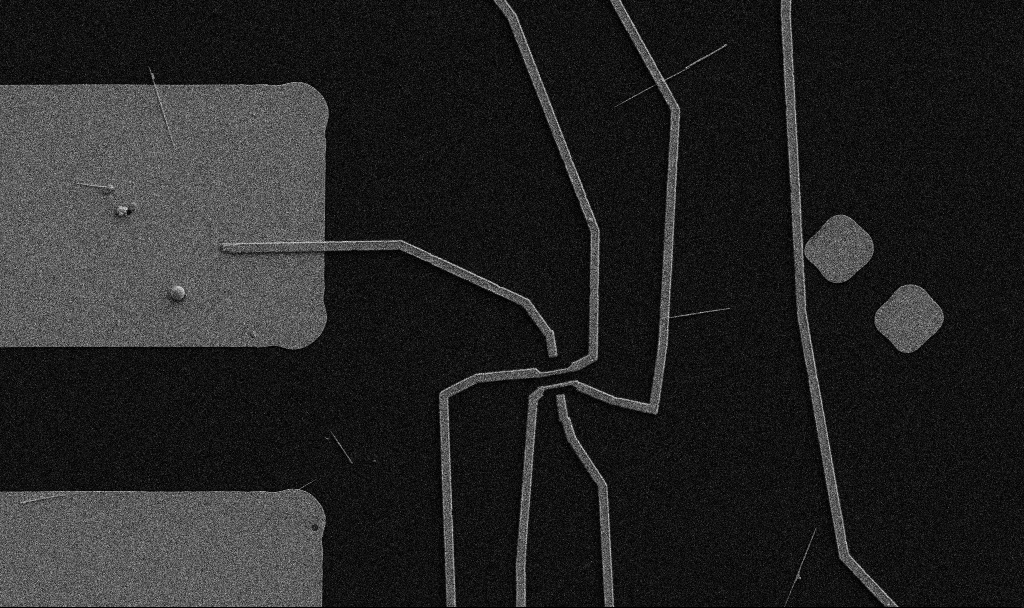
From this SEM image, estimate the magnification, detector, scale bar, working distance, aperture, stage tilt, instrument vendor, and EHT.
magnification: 5 K X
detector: SE2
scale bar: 10000 nm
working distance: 10.7 mm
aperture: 30 µm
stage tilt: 0°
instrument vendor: Zeiss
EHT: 5 kV